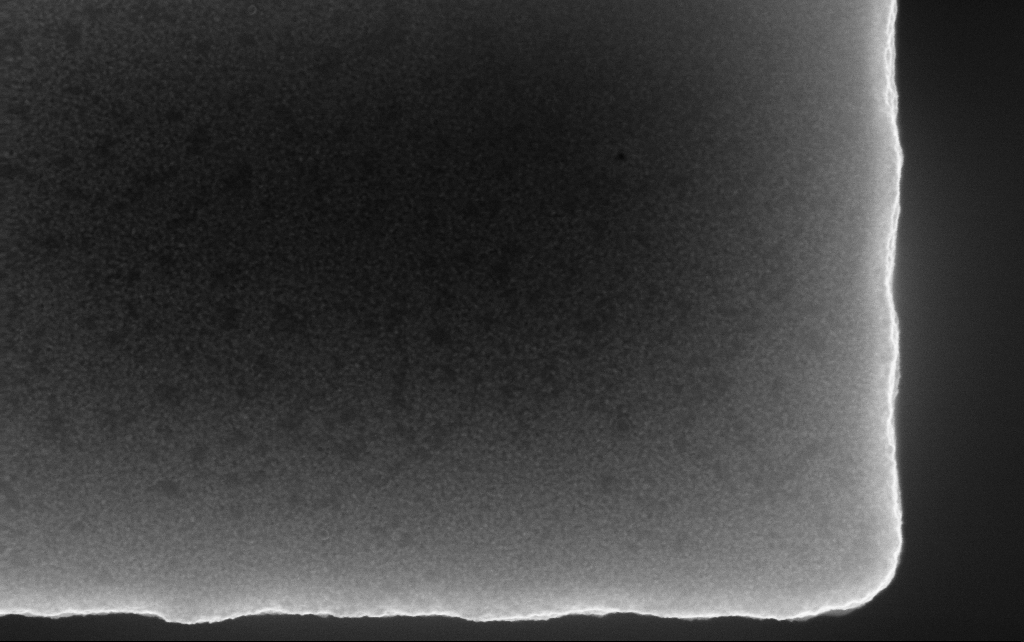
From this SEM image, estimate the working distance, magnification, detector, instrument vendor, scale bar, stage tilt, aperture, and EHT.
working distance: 2.5 mm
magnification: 143.51 K X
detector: InLens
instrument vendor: Zeiss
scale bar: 200 nm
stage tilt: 0°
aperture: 30 µm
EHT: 10 kV